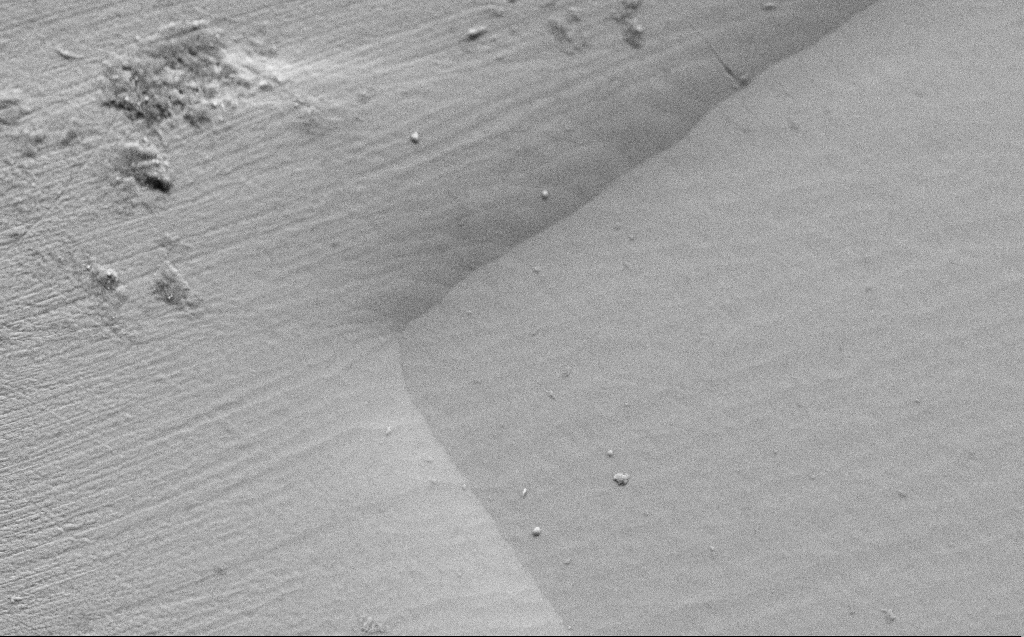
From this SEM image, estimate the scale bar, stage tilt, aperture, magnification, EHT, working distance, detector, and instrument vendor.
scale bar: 10000 nm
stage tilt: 45°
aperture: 30 µm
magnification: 4.82 K X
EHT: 5 kV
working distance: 8 mm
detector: SE2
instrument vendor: Zeiss